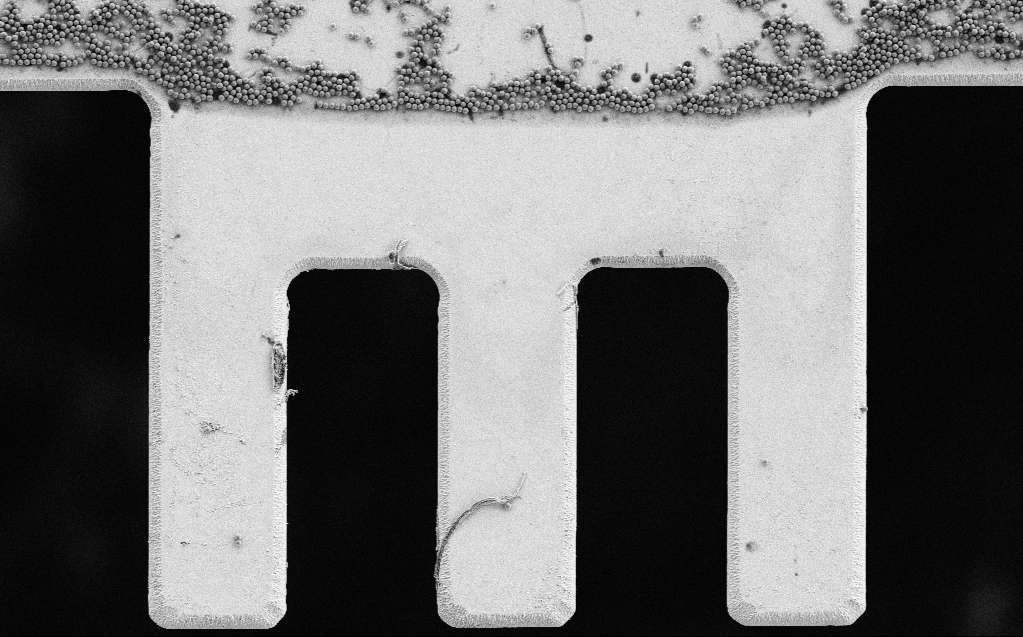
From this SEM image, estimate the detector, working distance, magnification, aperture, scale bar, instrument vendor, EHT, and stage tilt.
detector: SE2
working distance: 7 mm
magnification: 2.66 K X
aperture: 30 µm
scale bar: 20000 nm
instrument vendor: Zeiss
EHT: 3 kV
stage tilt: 0°